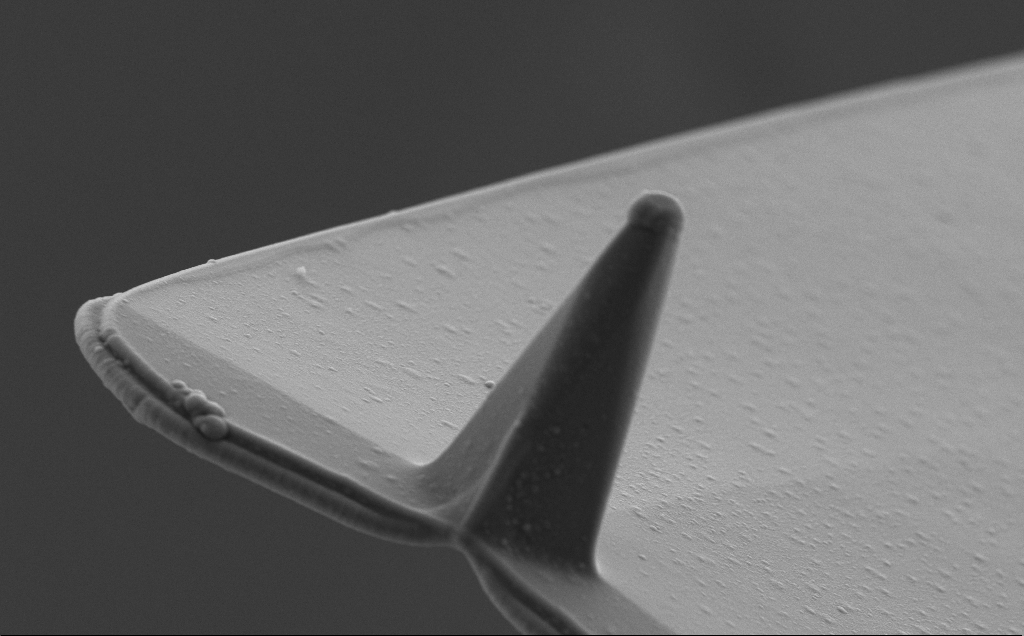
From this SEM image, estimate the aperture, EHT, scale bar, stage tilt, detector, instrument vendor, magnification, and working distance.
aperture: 30 µm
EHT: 5 kV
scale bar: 2000 nm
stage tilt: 30°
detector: SE2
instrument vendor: Zeiss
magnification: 11.7 K X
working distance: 8 mm